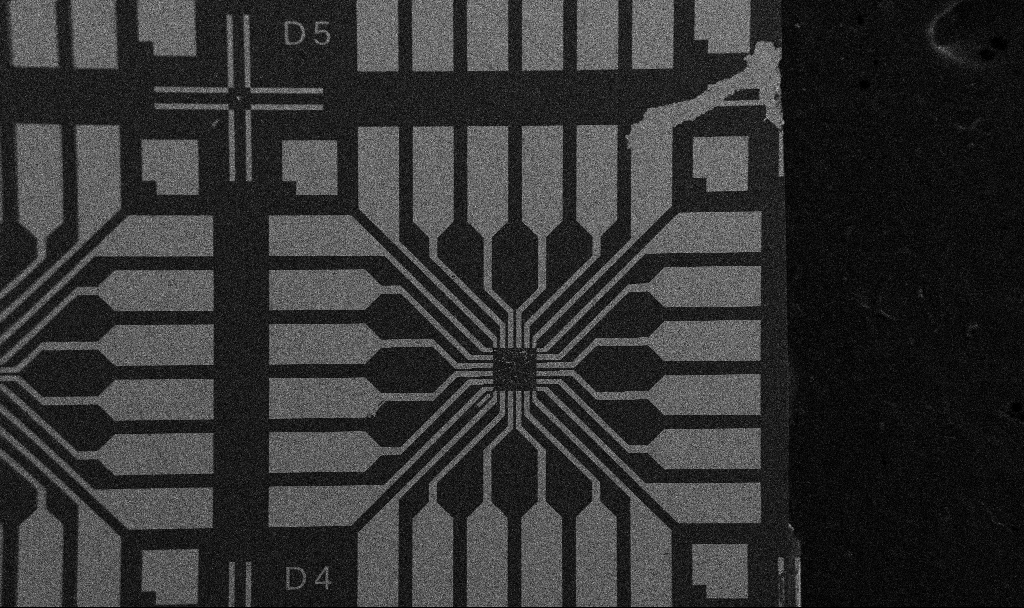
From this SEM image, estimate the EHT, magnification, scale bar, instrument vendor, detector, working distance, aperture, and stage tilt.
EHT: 5 kV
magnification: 0.1 K X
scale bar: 200000 nm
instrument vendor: Zeiss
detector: SE2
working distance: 10.7 mm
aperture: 30 µm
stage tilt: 0°